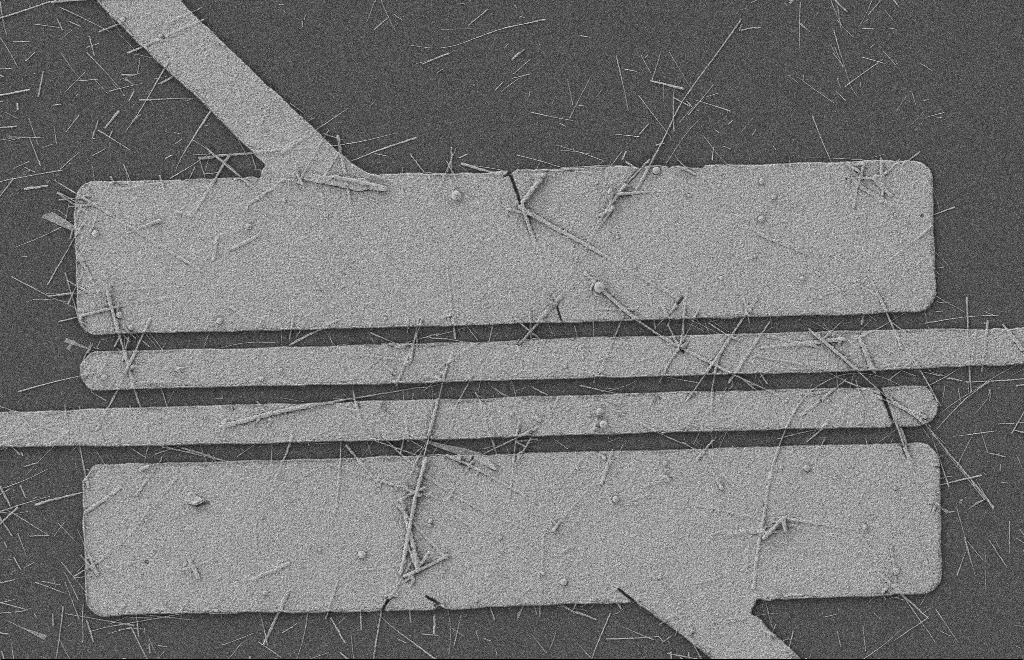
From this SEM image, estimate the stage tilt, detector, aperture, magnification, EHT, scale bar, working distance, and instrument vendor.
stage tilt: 0°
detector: SE2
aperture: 20 µm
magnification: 5.17 K X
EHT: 2 kV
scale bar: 2000 nm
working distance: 9 mm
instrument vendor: Zeiss